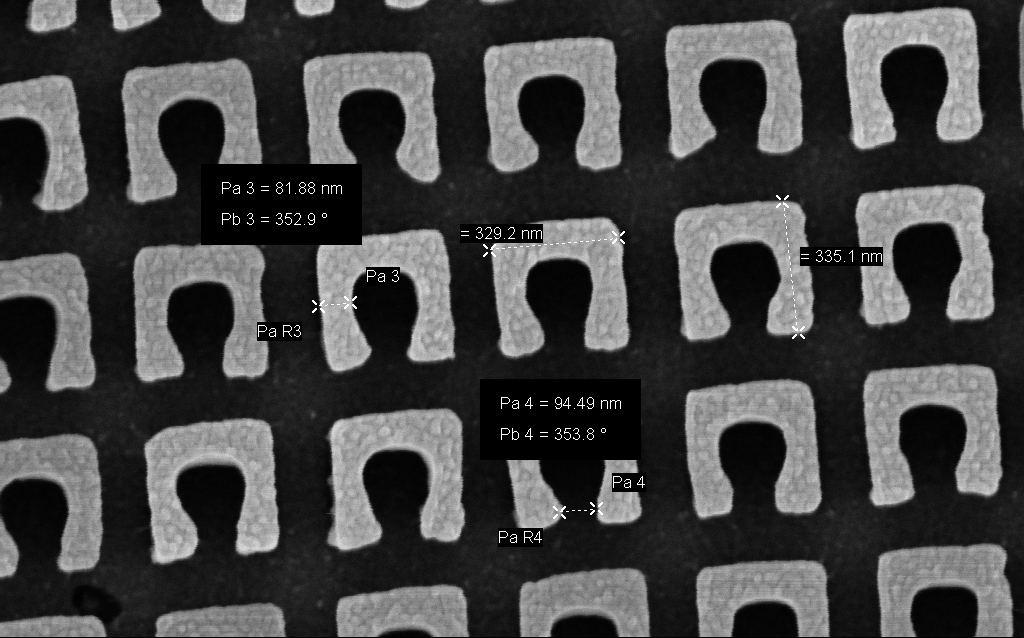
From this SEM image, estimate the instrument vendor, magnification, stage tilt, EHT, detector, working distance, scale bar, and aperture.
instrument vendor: Zeiss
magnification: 144.62 K X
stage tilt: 0°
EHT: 3 kV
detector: InLens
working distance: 4.6 mm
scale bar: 200 nm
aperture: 30 µm